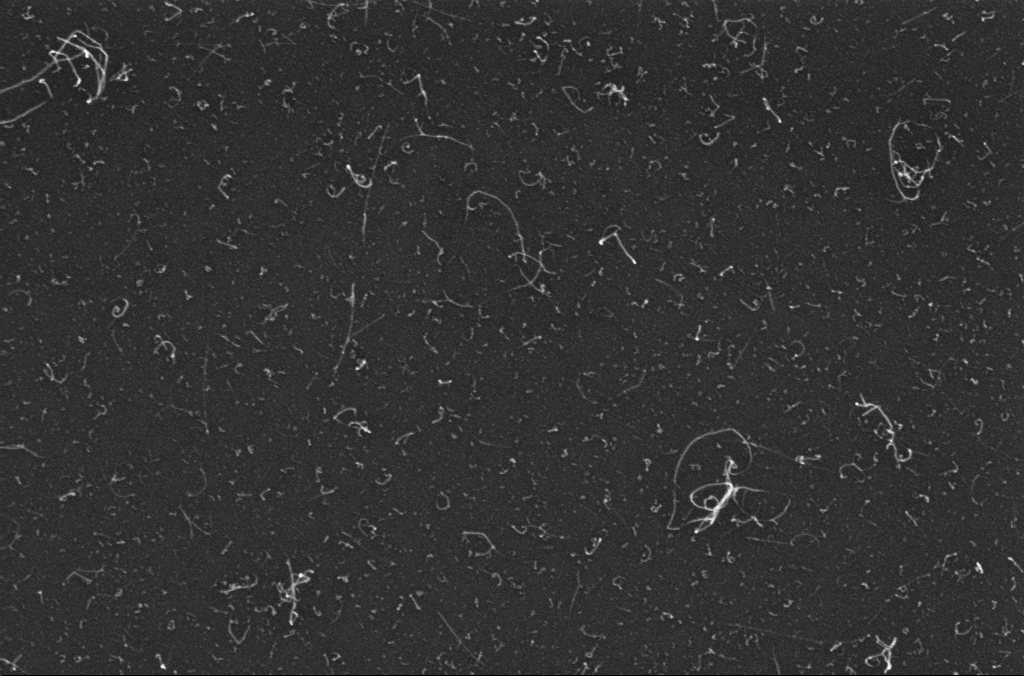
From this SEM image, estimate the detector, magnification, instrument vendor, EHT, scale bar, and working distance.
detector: InLens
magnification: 80 K X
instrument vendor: Zeiss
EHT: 10 kV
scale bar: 200 nm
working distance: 3.3 mm